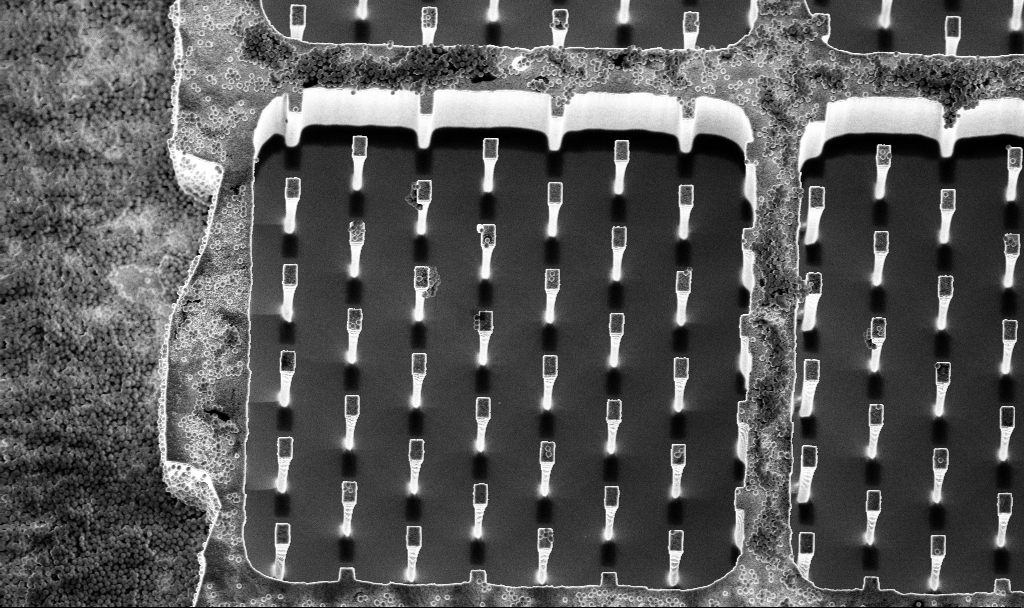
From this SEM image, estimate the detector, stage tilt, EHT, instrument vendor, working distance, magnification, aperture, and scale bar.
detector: InLens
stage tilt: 15°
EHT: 5 kV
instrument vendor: Zeiss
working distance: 3.2 mm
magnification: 3.39 K X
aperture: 30 µm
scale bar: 10000 nm